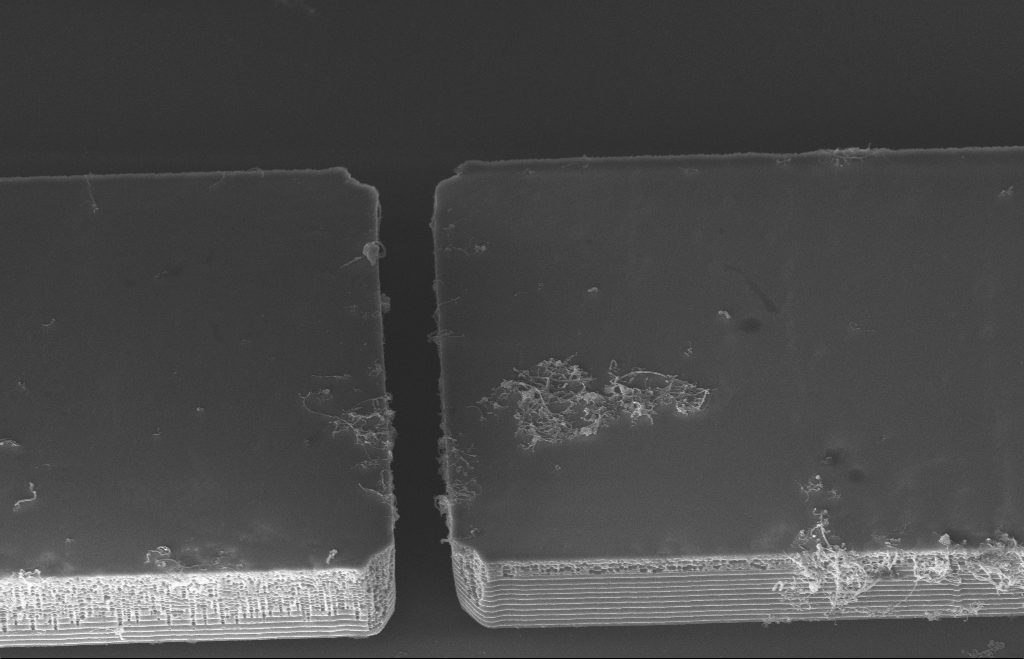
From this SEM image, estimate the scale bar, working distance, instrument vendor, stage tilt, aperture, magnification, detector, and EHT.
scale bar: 1000 nm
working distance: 9 mm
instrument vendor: Zeiss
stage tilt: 39°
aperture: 20 µm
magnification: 10.55 K X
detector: InLens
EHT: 5 kV